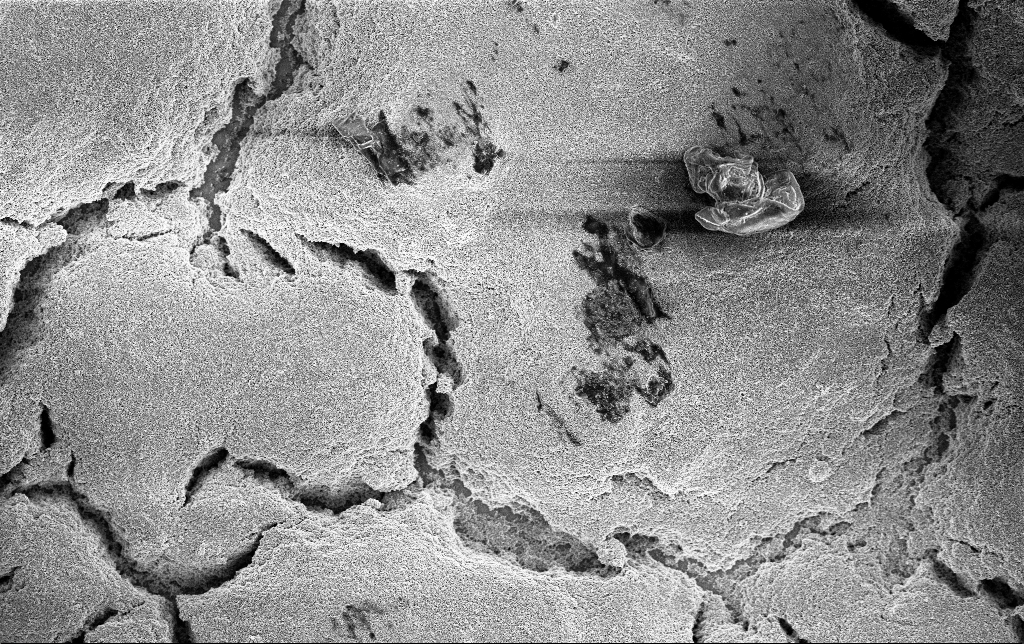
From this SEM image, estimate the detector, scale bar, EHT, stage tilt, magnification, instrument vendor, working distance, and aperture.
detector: InLens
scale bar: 10000 nm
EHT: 3 kV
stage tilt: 0°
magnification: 3 K X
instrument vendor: Zeiss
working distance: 2.8 mm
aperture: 30 µm